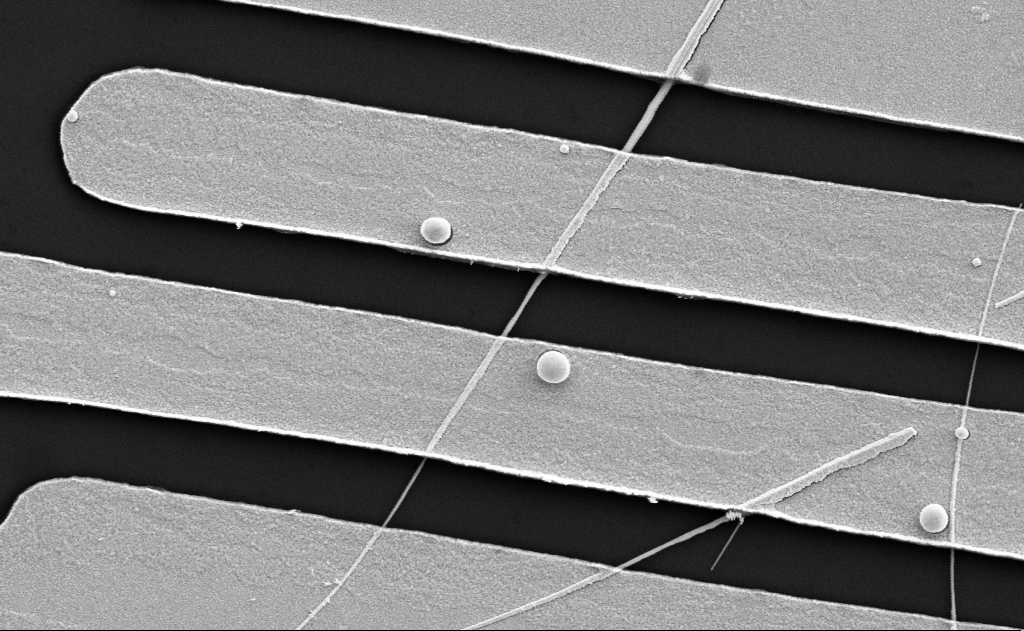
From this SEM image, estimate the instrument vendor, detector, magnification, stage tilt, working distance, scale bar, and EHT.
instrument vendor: Zeiss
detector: SE2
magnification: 19.71 K X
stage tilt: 0°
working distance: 19 mm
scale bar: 2000 nm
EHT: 5 kV